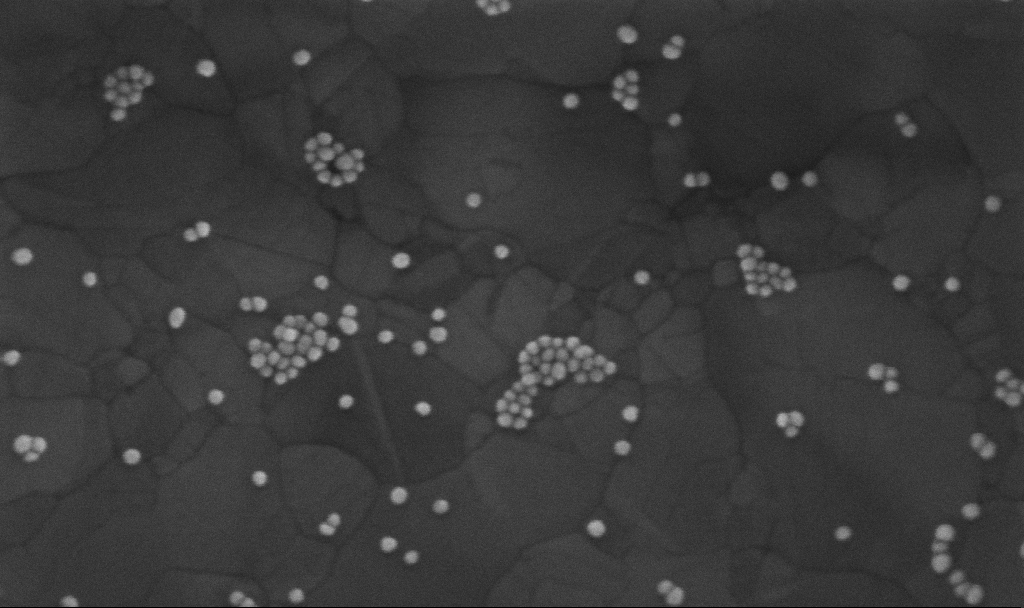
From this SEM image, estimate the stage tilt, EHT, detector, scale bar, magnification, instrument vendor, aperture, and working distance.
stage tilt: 0°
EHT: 10 kV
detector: InLens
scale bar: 200 nm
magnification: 300 K X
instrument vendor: Zeiss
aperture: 30 µm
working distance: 3.8 mm